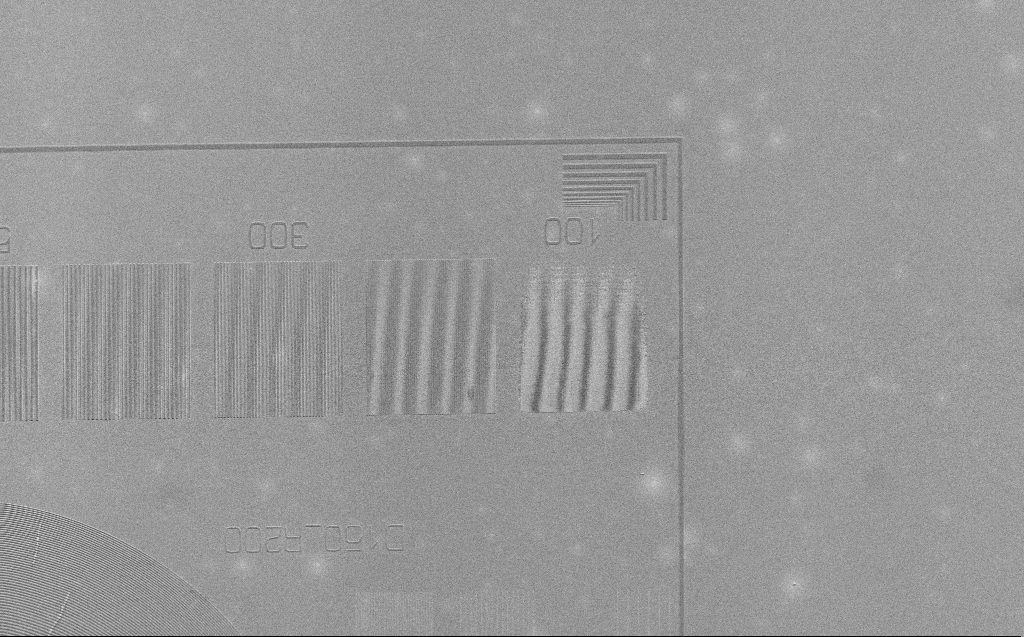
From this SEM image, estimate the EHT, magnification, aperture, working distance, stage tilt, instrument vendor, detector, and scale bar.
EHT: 2.5 kV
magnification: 1.94 K X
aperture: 30 µm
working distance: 5 mm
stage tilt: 30°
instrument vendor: Zeiss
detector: SE2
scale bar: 10000 nm